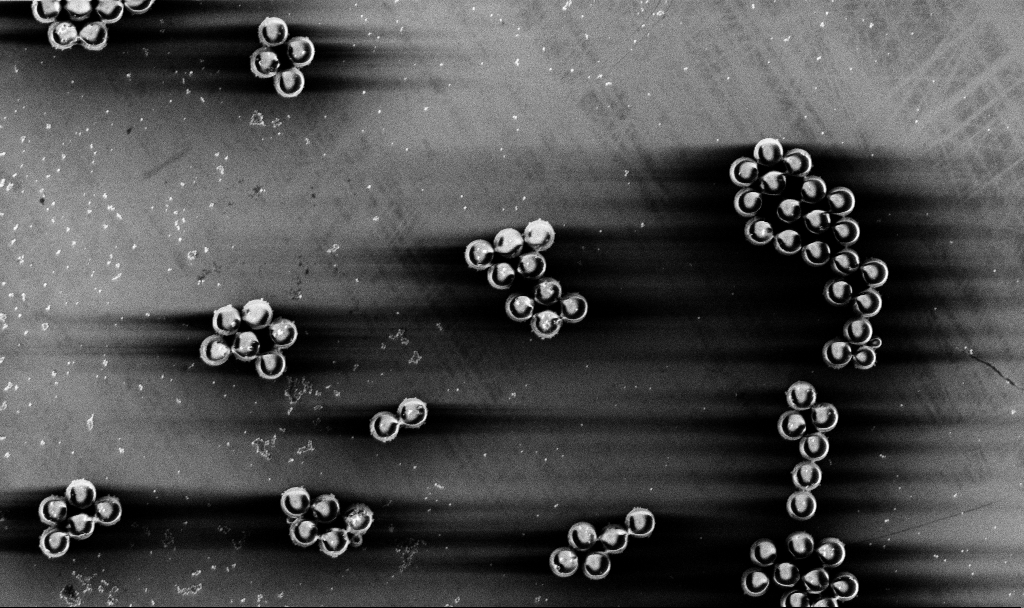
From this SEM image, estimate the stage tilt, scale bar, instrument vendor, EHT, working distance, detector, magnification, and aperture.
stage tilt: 0°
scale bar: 10000 nm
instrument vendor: Zeiss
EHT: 3 kV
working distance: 5.2 mm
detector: InLens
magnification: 5.42 K X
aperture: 30 µm